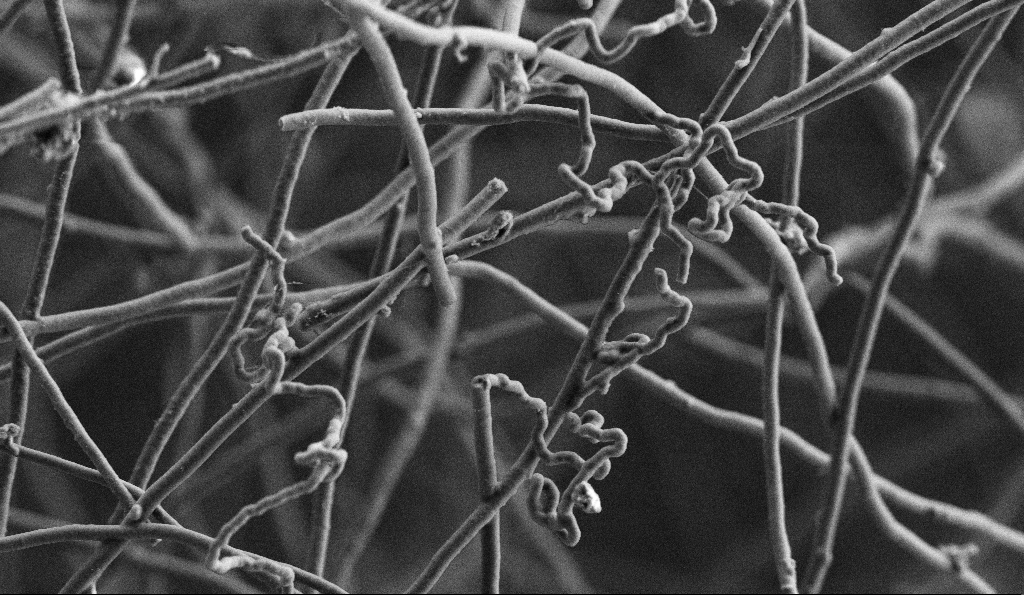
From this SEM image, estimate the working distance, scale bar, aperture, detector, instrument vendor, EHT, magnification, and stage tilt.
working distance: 5.5 mm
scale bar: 2000 nm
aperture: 30 µm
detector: SE2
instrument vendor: Zeiss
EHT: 3 kV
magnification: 10 K X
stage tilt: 0°